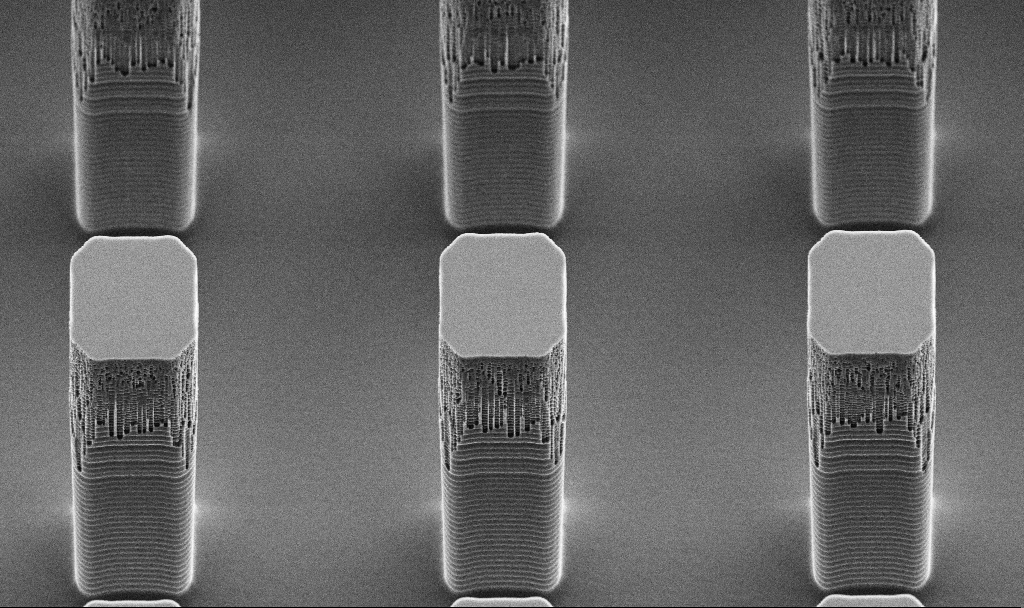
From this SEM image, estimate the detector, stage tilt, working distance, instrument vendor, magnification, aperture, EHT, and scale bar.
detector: SE2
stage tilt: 45°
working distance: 11.6 mm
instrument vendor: Zeiss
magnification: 4.49 K X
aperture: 30 µm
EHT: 5 kV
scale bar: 10000 nm